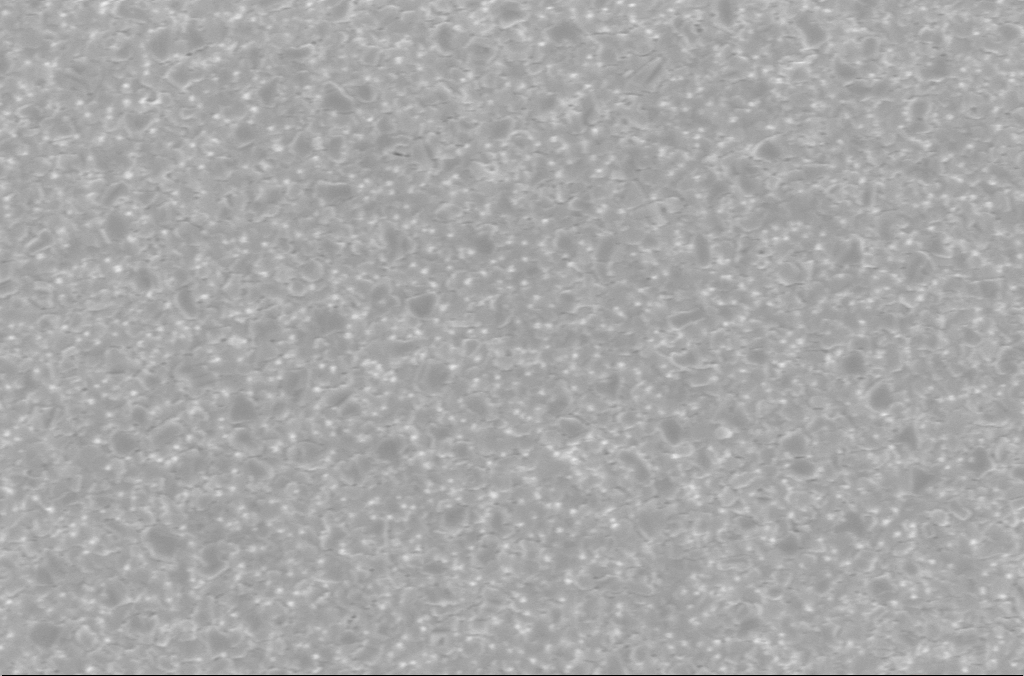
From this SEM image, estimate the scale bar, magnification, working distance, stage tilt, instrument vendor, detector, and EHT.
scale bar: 2000 nm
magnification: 20 K X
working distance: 3 mm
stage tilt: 0°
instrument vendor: Zeiss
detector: InLens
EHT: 5 kV